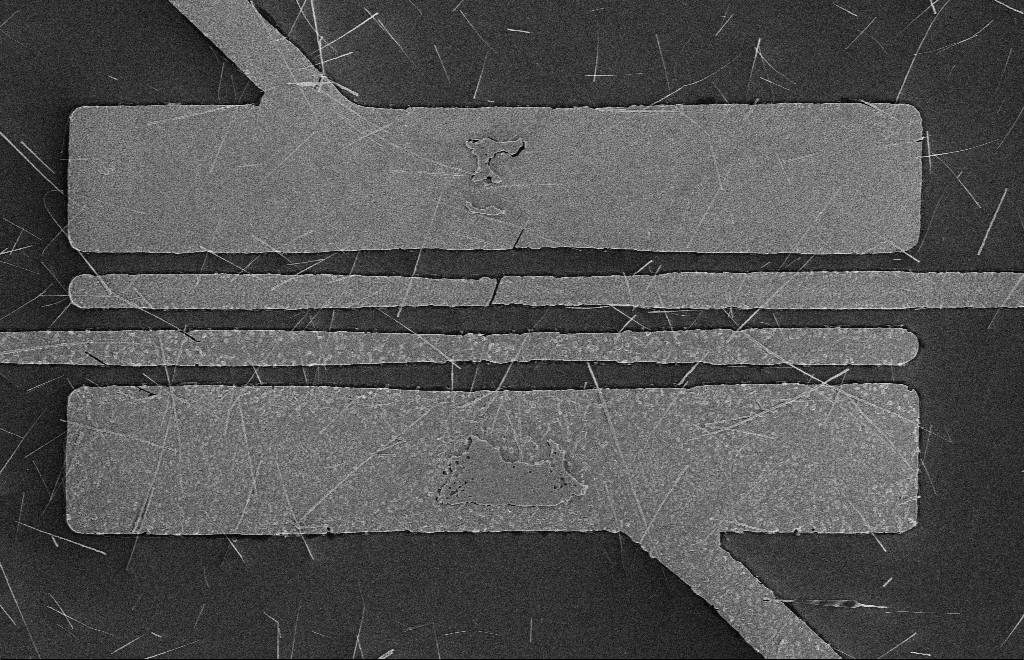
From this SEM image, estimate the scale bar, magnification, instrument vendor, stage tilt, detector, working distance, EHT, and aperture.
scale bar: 2000 nm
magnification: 5.17 K X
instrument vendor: Zeiss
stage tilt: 0°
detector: SE2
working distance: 16 mm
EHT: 5 kV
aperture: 10 µm